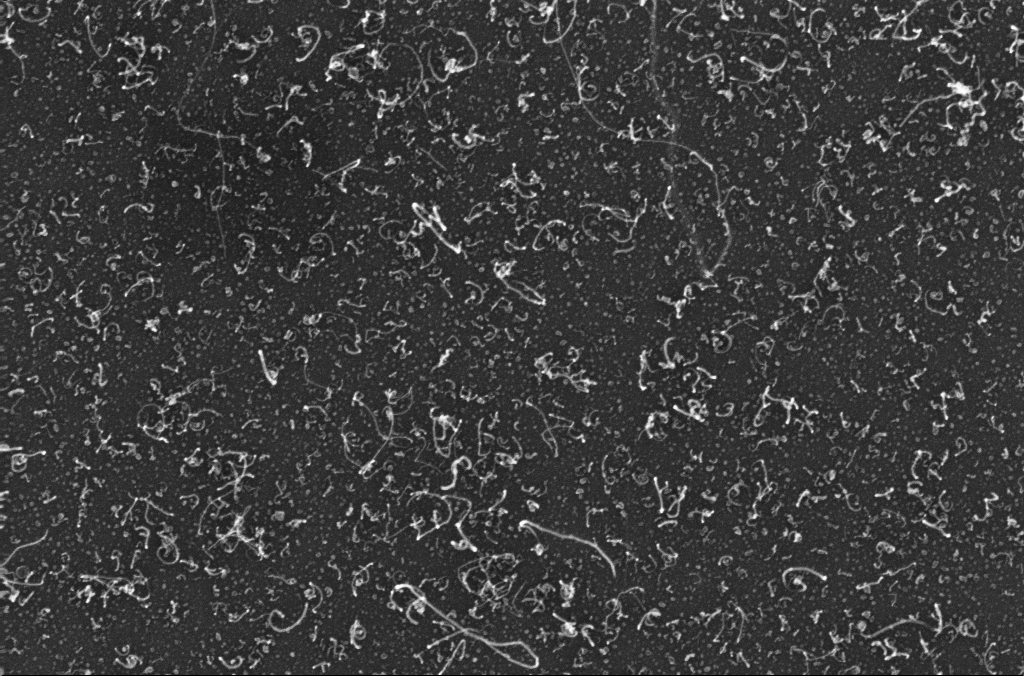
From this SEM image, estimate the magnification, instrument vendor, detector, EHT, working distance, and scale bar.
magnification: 100 K X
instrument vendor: Zeiss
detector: InLens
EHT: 10 kV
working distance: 3.3 mm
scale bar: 200 nm